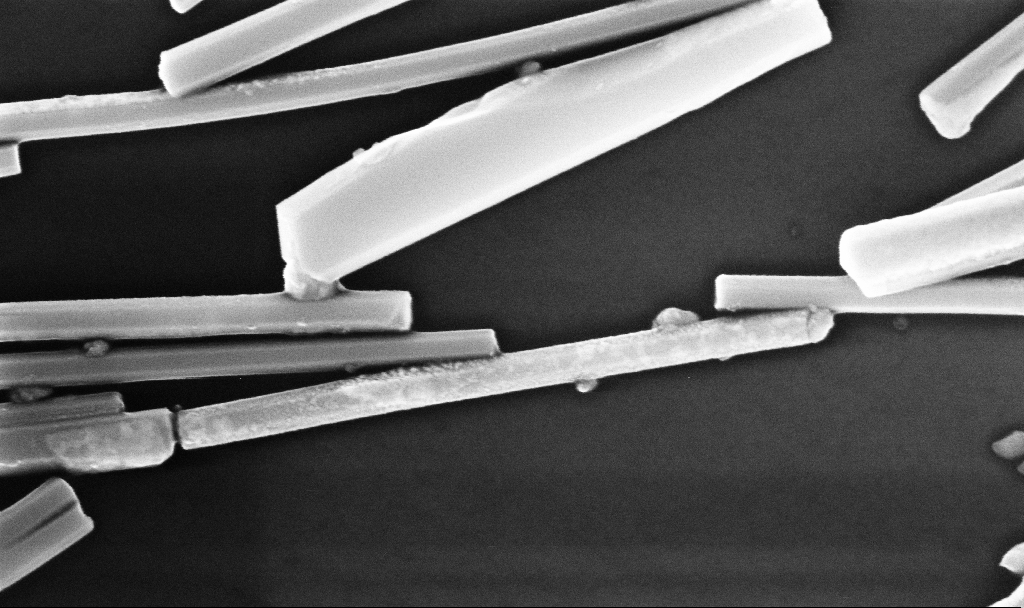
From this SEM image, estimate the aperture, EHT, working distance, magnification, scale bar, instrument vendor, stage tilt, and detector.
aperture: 30 µm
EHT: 10 kV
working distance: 6.7 mm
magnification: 123.11 K X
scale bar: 200 nm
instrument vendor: Zeiss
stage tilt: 0°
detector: InLens